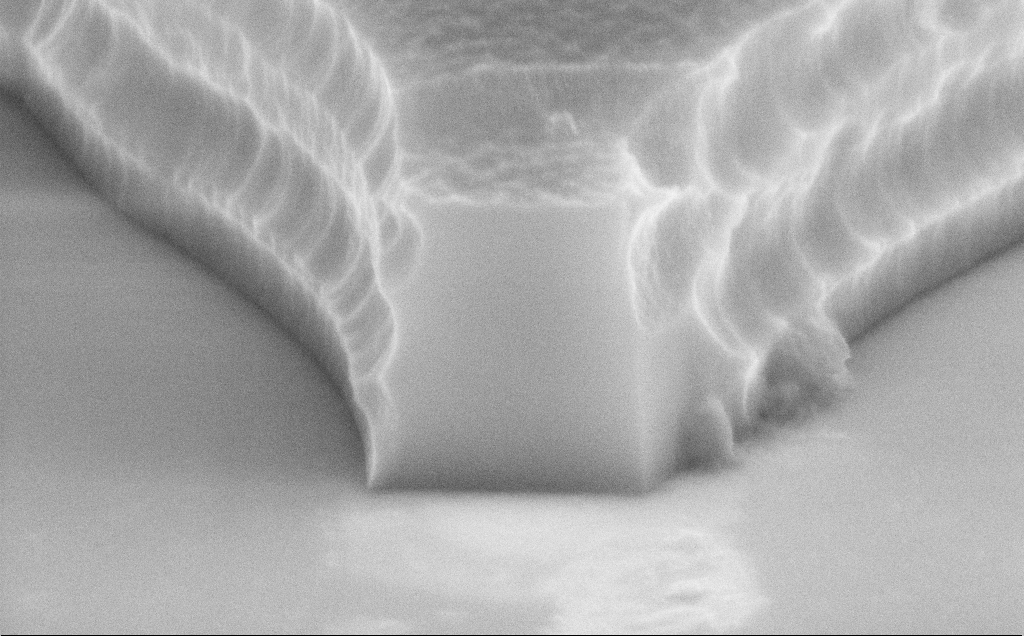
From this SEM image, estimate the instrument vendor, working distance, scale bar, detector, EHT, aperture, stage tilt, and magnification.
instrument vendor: Zeiss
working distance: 12 mm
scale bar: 1000 nm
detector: InLens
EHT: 8 kV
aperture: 30 µm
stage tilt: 70°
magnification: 65.5 K X